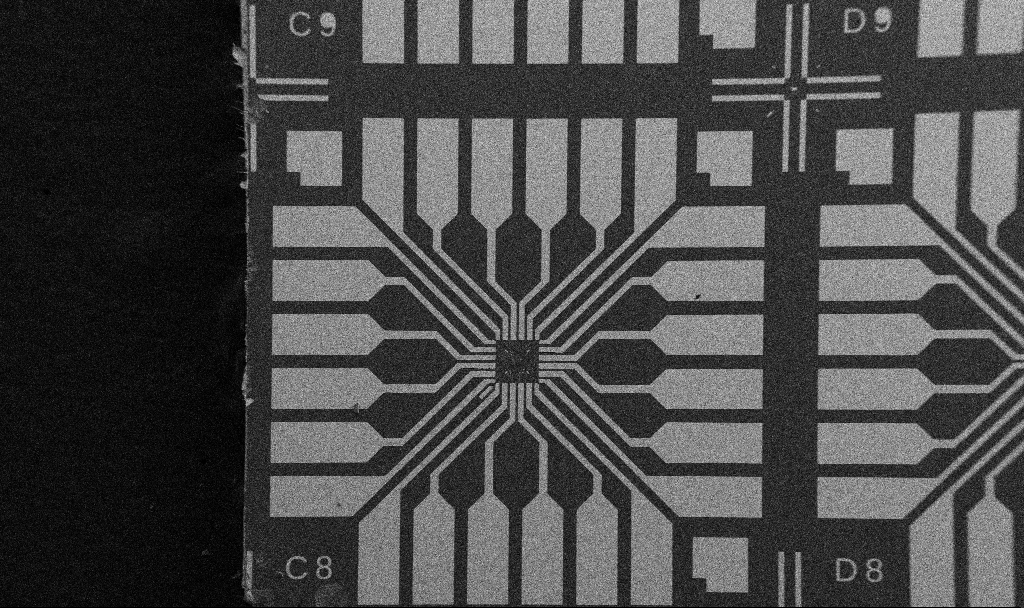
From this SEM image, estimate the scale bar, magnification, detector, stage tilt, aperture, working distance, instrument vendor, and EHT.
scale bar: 200000 nm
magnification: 0.1 K X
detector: SE2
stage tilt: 0°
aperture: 30 µm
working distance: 10.7 mm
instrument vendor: Zeiss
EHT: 5 kV